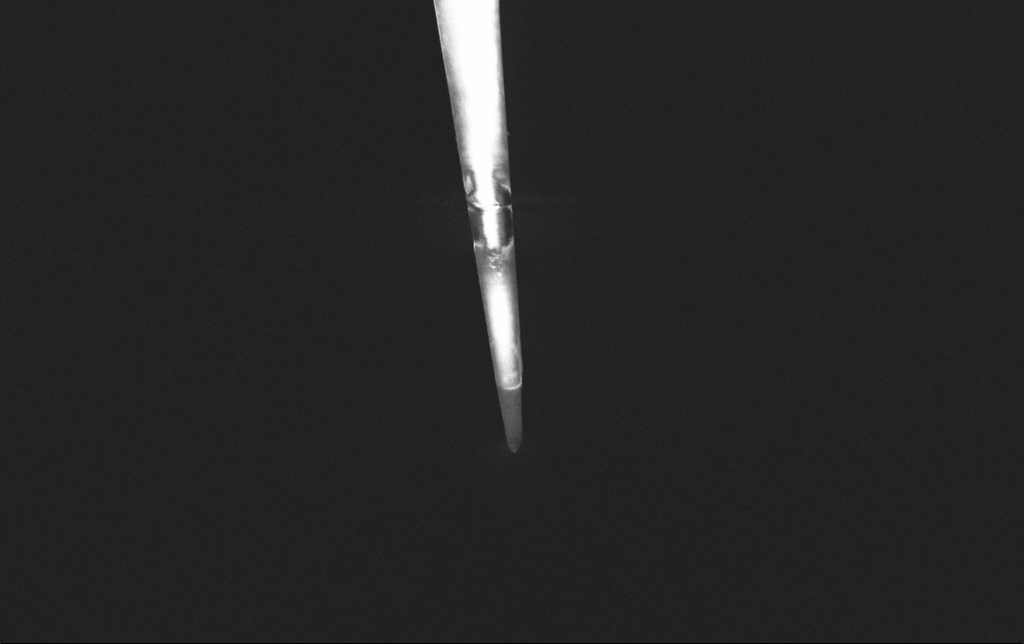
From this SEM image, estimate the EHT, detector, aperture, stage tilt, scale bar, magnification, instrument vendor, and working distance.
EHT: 3 kV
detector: InLens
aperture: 30 µm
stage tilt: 0°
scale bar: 10000 nm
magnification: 5 K X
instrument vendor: Zeiss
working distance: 6.5 mm